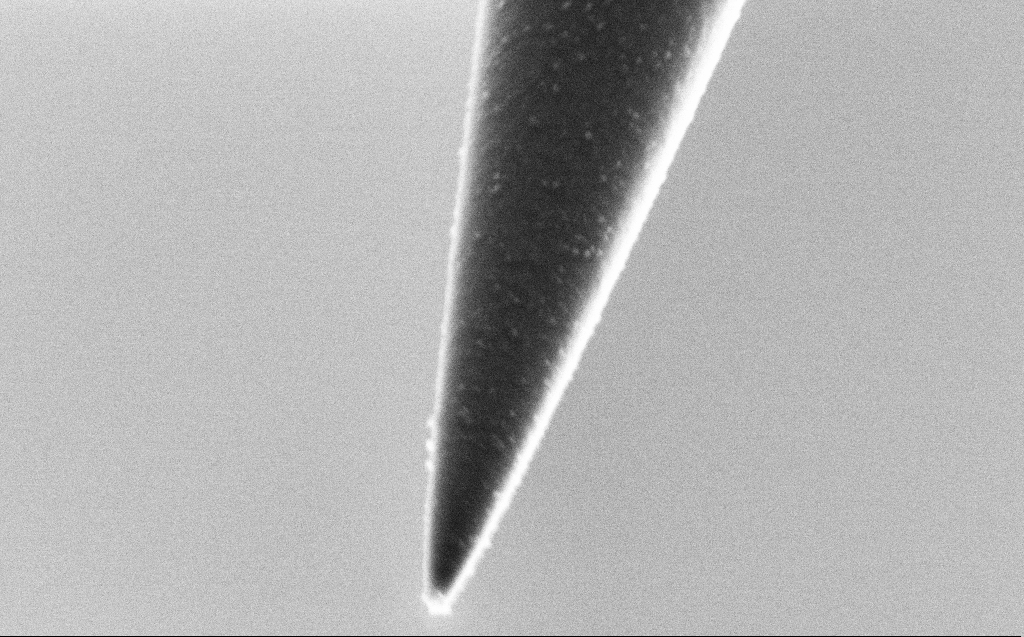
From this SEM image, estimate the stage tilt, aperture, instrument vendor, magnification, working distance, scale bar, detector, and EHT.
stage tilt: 45°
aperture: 30 µm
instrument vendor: Zeiss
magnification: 85.25 K X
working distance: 5 mm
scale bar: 200 nm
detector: SE2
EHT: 2 kV